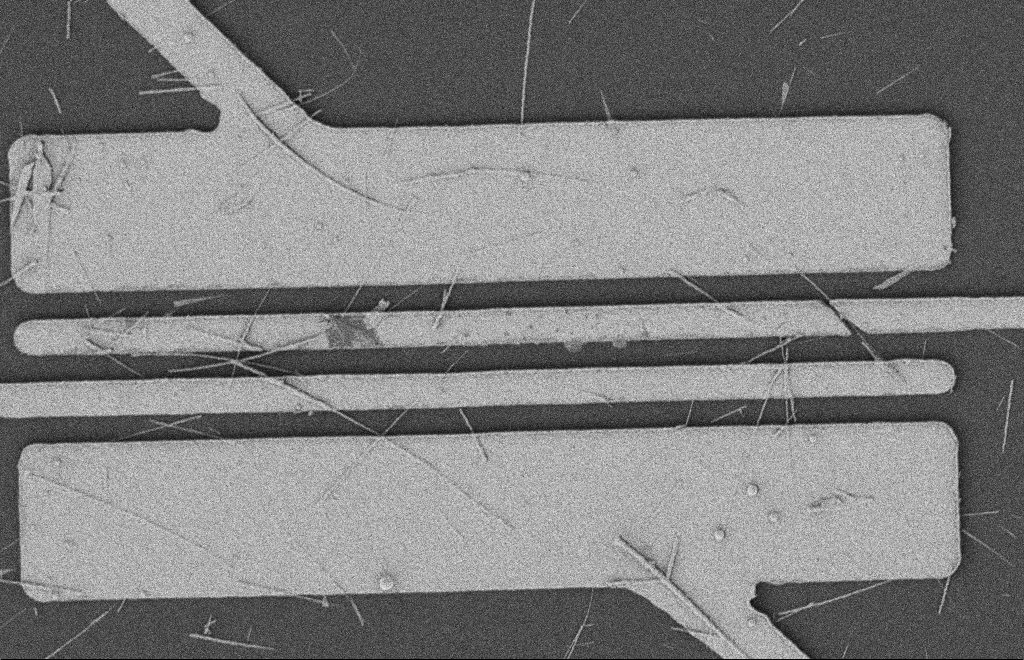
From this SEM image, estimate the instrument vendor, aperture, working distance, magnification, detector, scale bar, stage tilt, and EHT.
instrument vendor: Zeiss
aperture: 20 µm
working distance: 12 mm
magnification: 5.61 K X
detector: SE2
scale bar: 2000 nm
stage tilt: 0°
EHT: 2 kV